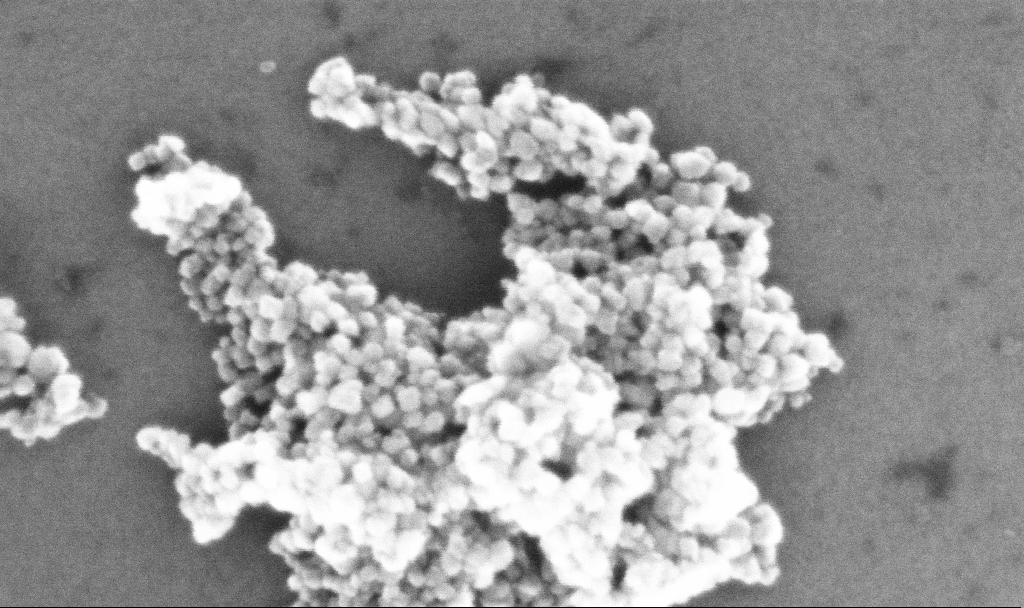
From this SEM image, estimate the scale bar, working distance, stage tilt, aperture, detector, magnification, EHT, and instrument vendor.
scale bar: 100 nm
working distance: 5.2 mm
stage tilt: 0°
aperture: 30 µm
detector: InLens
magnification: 434.61 K X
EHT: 10 kV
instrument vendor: Zeiss